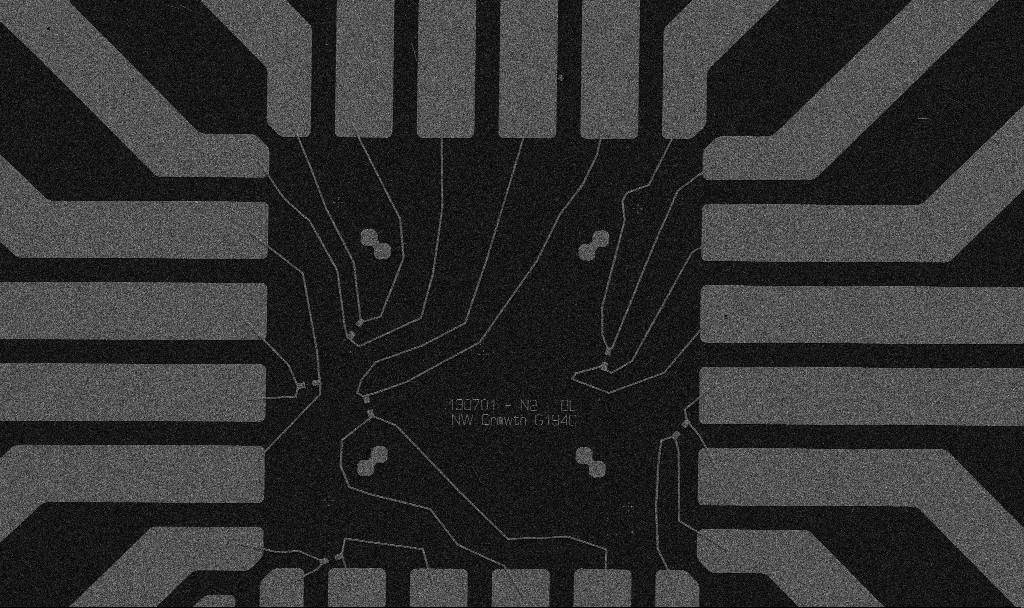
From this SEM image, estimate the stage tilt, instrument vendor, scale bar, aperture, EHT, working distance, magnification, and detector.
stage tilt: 0°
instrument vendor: Zeiss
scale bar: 20000 nm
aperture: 30 µm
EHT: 5 kV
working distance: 10.7 mm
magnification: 1 K X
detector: SE2